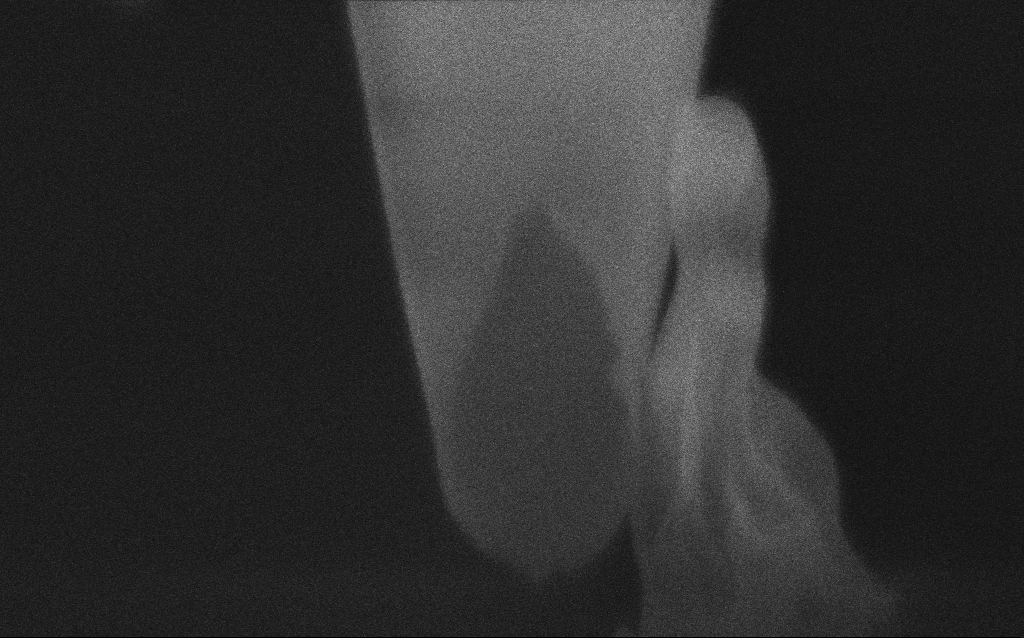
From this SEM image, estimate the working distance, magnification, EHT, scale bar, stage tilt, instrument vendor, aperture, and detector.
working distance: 4 mm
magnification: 221.93 K X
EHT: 2 kV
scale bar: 200 nm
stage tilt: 45°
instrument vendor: Zeiss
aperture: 30 µm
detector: InLens